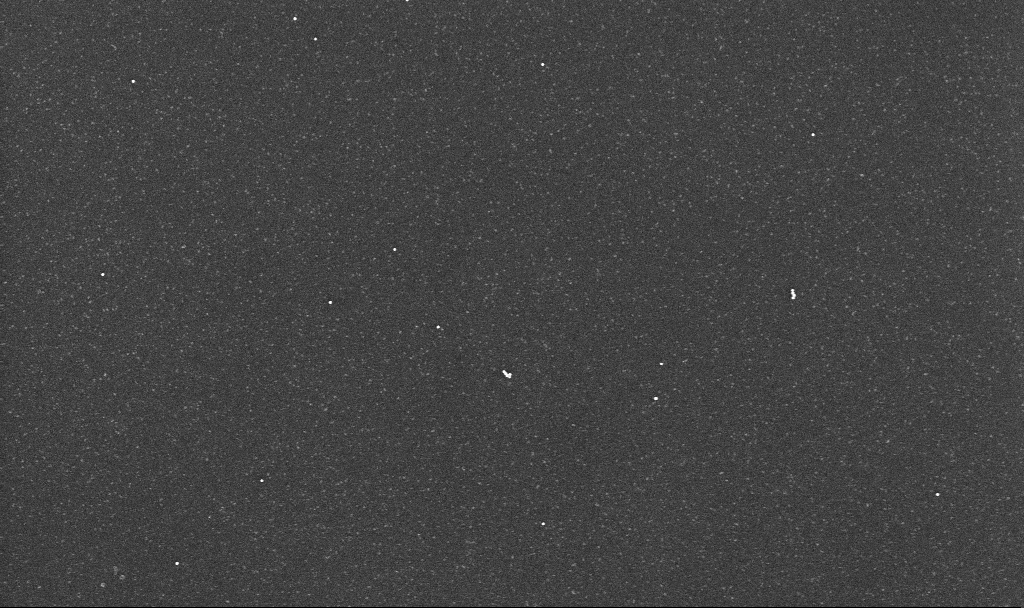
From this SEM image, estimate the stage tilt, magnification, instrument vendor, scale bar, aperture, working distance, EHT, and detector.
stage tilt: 0°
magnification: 43.83 K X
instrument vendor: Zeiss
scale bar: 1000 nm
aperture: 30 µm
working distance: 3.1 mm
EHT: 10 kV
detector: InLens